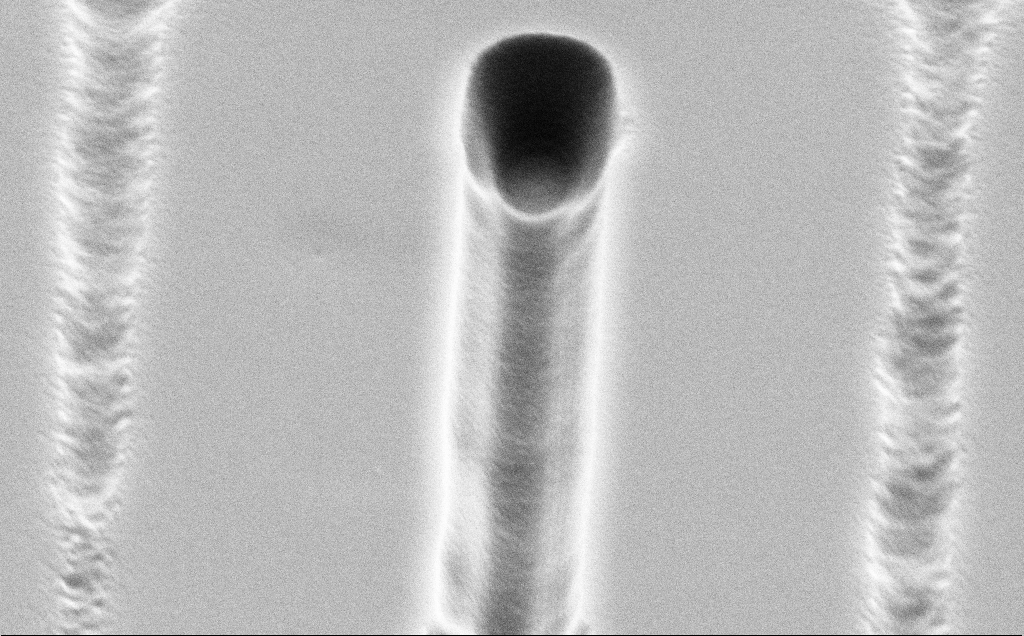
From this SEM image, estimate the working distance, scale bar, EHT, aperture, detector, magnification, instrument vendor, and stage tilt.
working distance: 11 mm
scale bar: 2000 nm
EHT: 5 kV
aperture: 30 µm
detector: SE2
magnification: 29.65 K X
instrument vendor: Zeiss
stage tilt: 45°